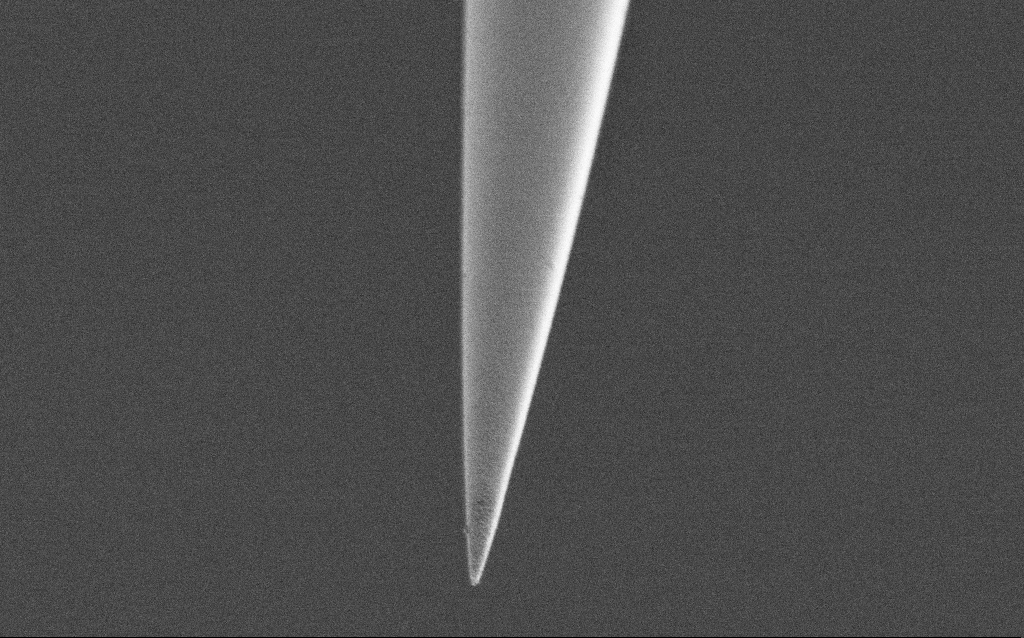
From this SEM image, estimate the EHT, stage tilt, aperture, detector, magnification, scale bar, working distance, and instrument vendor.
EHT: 1 kV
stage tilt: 45°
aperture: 30 µm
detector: SE2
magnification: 25 K X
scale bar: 1000 nm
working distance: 6.9 mm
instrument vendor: Zeiss